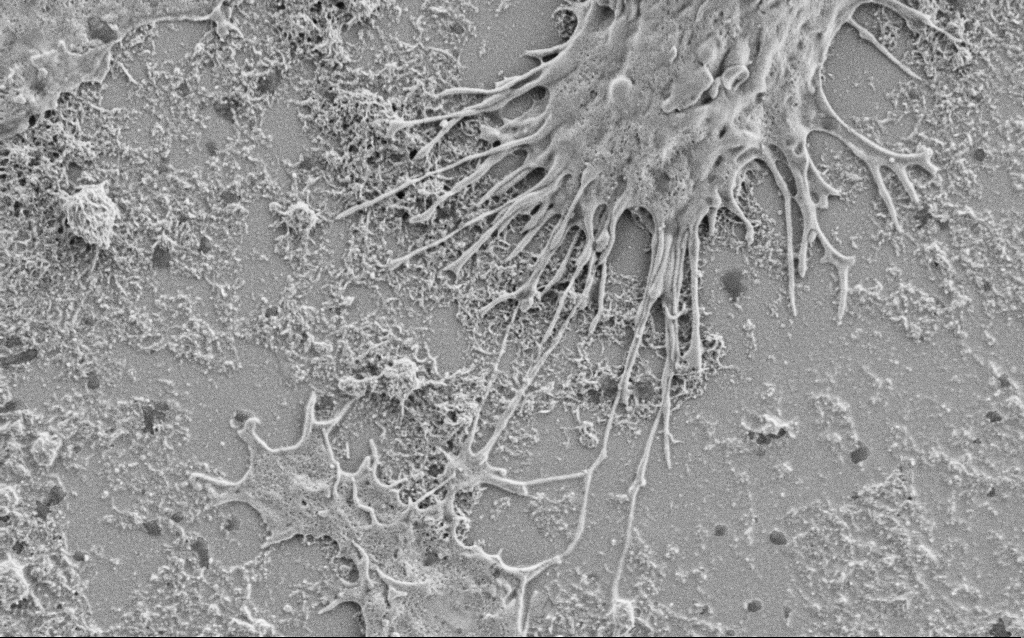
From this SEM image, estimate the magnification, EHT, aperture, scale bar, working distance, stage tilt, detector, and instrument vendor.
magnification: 10 K X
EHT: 2 kV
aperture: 30 µm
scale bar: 2000 nm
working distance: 6.9 mm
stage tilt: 0°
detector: SE2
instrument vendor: Zeiss